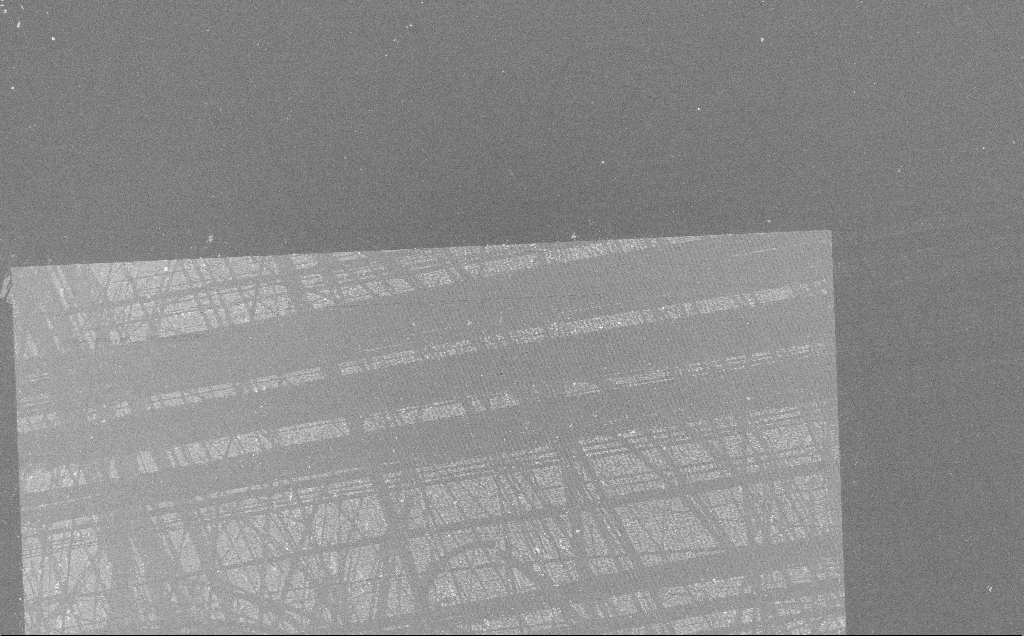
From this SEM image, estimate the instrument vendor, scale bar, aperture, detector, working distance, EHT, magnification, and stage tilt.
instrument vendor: Zeiss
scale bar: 100000 nm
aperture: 30 µm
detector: InLens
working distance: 7 mm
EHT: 10 kV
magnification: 0.304 K X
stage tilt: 0°